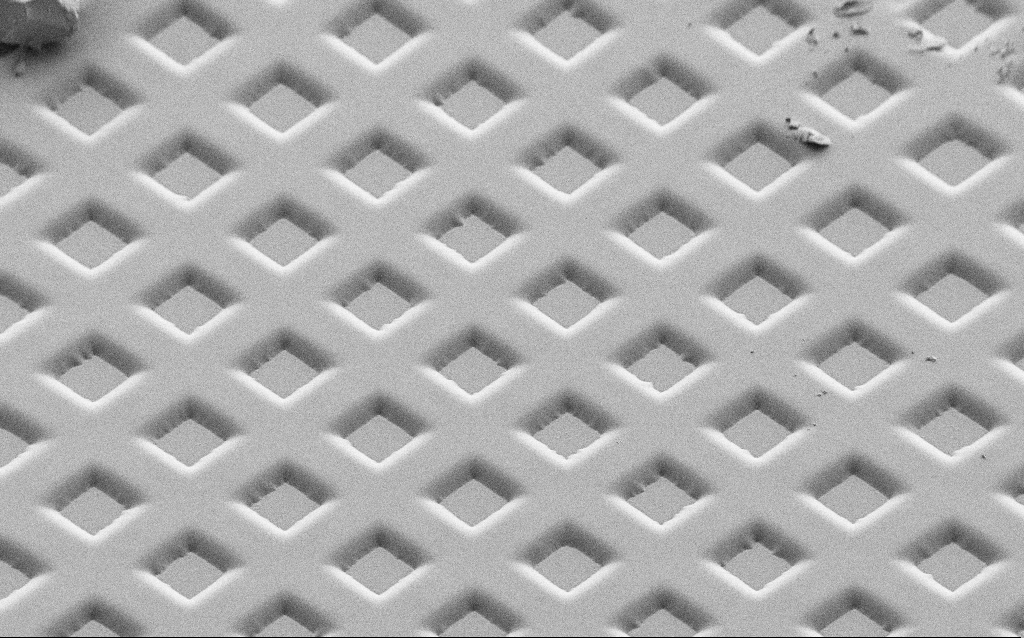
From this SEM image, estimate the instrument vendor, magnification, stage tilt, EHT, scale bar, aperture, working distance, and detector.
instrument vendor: Zeiss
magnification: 0.345 K X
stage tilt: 45°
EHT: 1.5 kV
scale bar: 100000 nm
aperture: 30 µm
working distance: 6 mm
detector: SE2